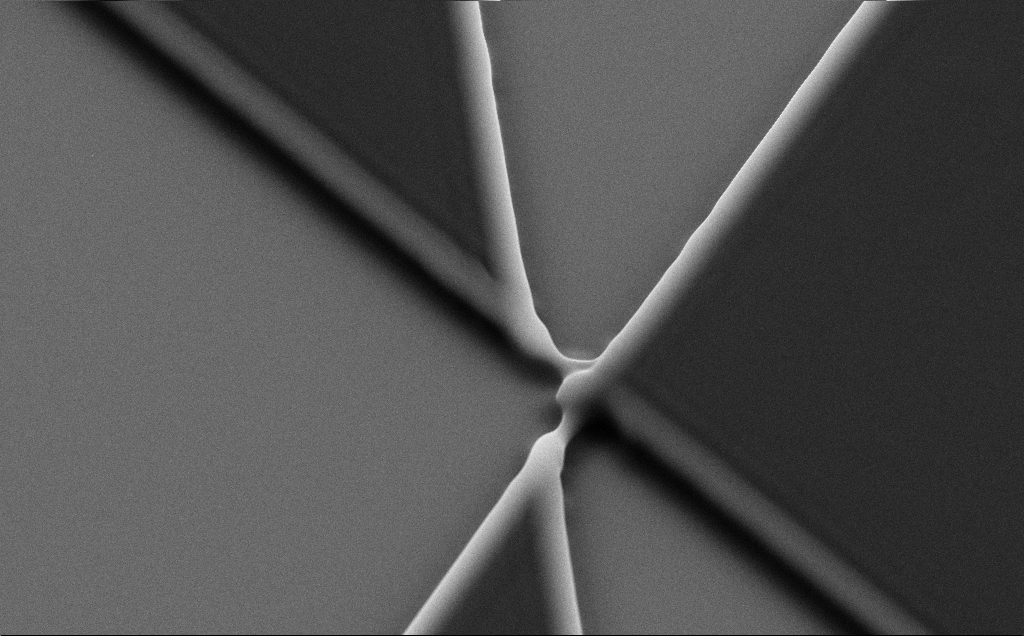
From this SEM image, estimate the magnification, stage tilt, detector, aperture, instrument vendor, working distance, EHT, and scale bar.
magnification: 9.43 K X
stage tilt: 35°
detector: SE2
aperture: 30 µm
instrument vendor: Zeiss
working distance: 8 mm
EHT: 10 kV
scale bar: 2000 nm